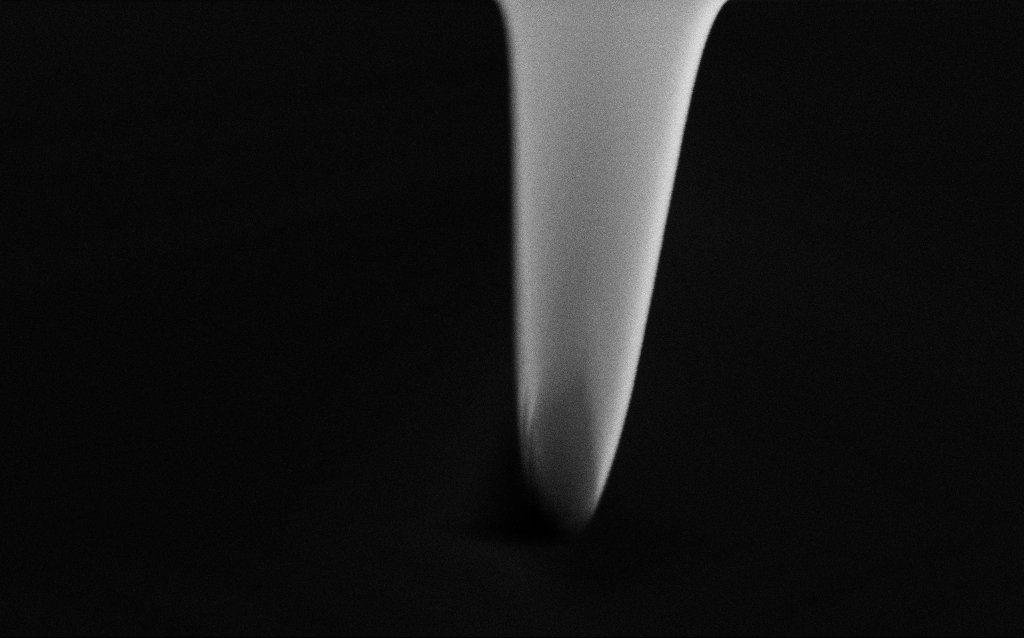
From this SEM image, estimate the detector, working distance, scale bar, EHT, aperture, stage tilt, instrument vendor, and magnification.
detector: SE2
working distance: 5 mm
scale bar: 200 nm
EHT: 5 kV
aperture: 30 µm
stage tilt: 45°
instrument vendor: Zeiss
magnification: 100.68 K X